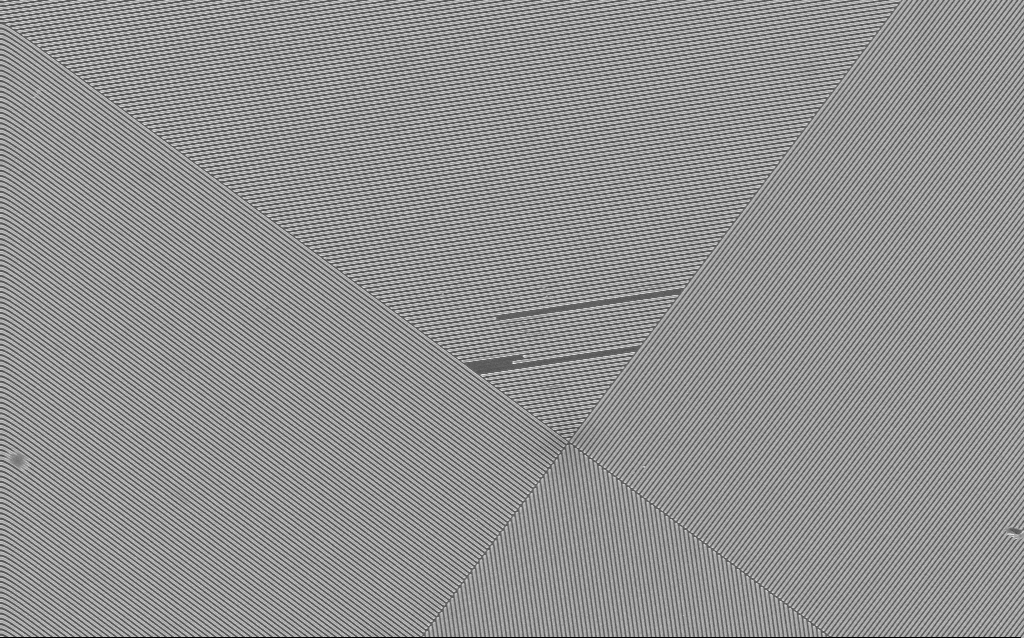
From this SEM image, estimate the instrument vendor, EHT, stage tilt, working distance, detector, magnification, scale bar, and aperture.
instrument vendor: Zeiss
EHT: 10 kV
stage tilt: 0°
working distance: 3.9 mm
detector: InLens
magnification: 3.89 K X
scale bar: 10000 nm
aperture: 30 µm